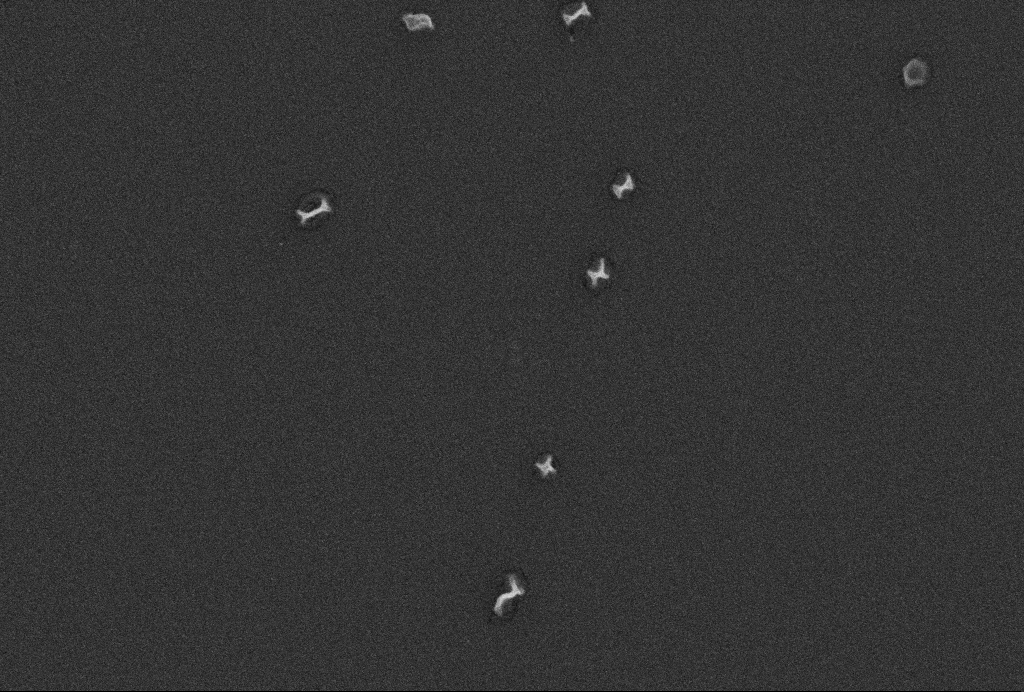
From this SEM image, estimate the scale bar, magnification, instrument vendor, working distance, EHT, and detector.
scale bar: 200 nm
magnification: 100 K X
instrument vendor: Zeiss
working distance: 2.6 mm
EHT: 5 kV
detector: SE2